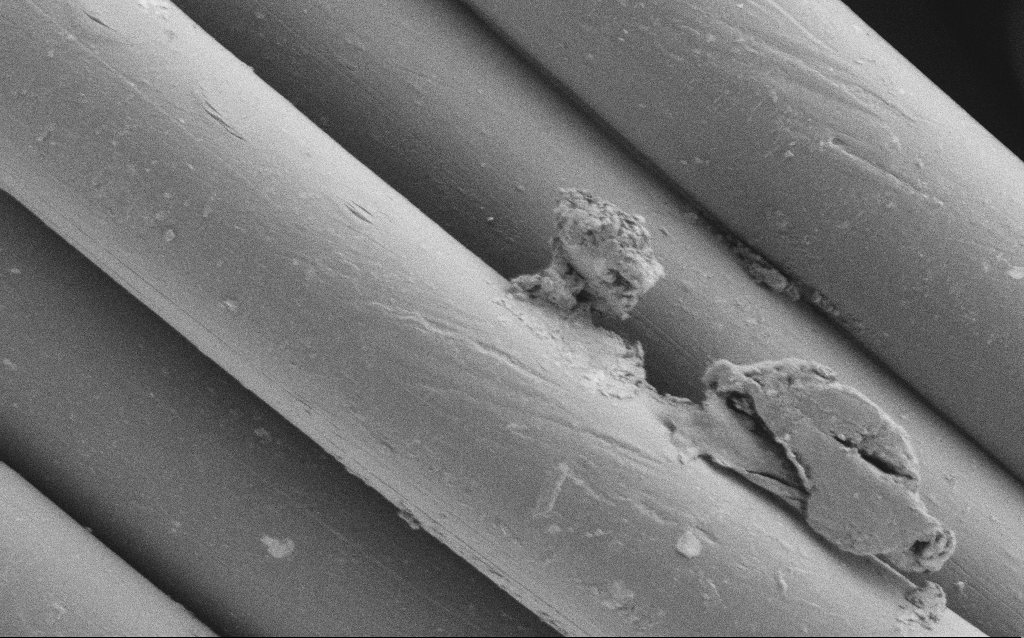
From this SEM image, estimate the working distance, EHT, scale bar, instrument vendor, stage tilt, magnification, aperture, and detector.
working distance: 4 mm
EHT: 1 kV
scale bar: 10000 nm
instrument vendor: Zeiss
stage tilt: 0°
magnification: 3.88 K X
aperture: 30 µm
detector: SE2